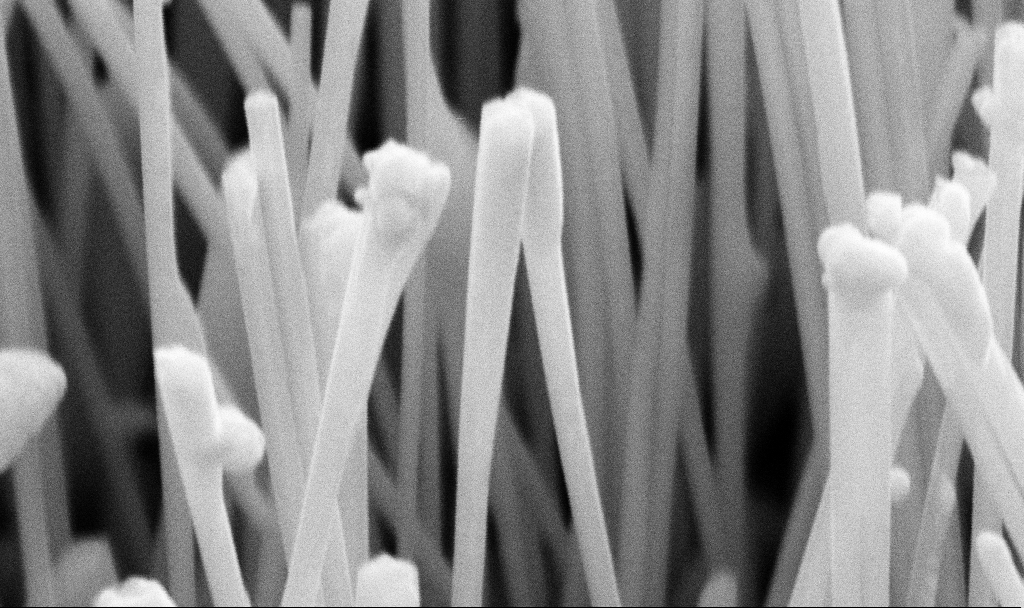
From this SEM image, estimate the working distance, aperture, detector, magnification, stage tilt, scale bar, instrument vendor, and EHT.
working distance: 11.2 mm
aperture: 30 µm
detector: SE2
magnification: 105.64 K X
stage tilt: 45°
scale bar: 200 nm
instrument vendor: Zeiss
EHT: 10 kV